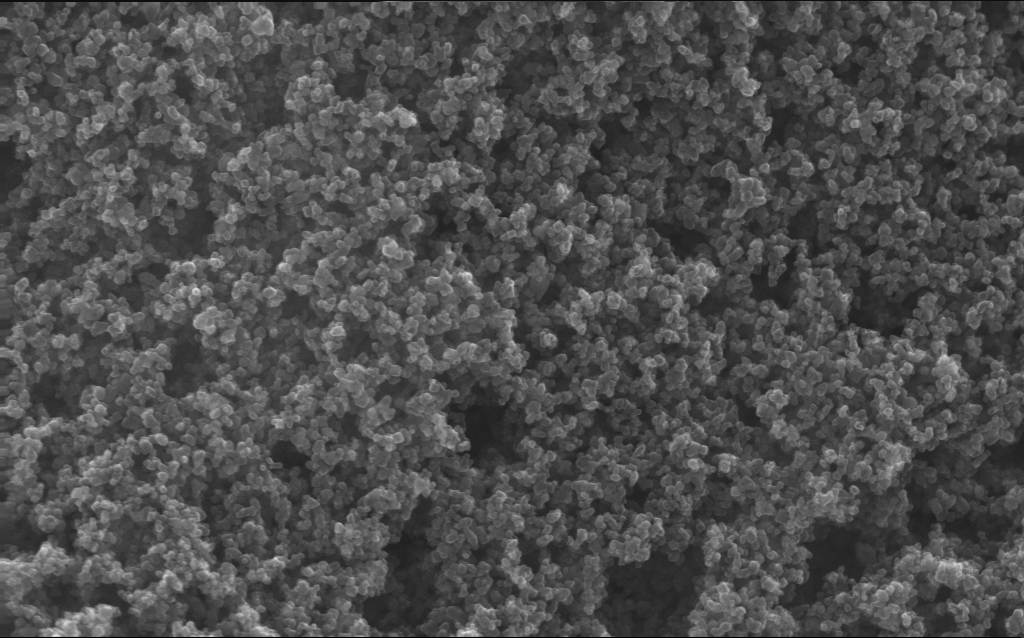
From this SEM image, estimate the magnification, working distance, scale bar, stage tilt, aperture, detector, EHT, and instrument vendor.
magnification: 130 K X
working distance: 3 mm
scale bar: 100 nm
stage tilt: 0°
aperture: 30 µm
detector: InLens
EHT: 20 kV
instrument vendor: Zeiss